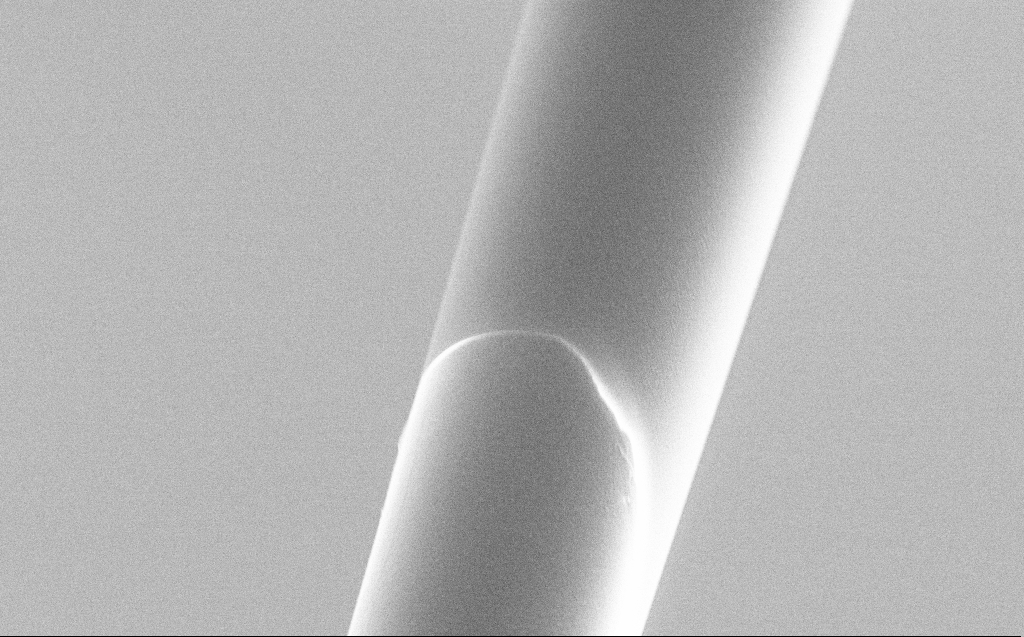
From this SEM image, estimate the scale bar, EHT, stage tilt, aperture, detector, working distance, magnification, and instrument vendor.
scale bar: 10000 nm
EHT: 5 kV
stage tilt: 45°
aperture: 30 µm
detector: SE2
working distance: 6 mm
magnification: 4.3 K X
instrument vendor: Zeiss